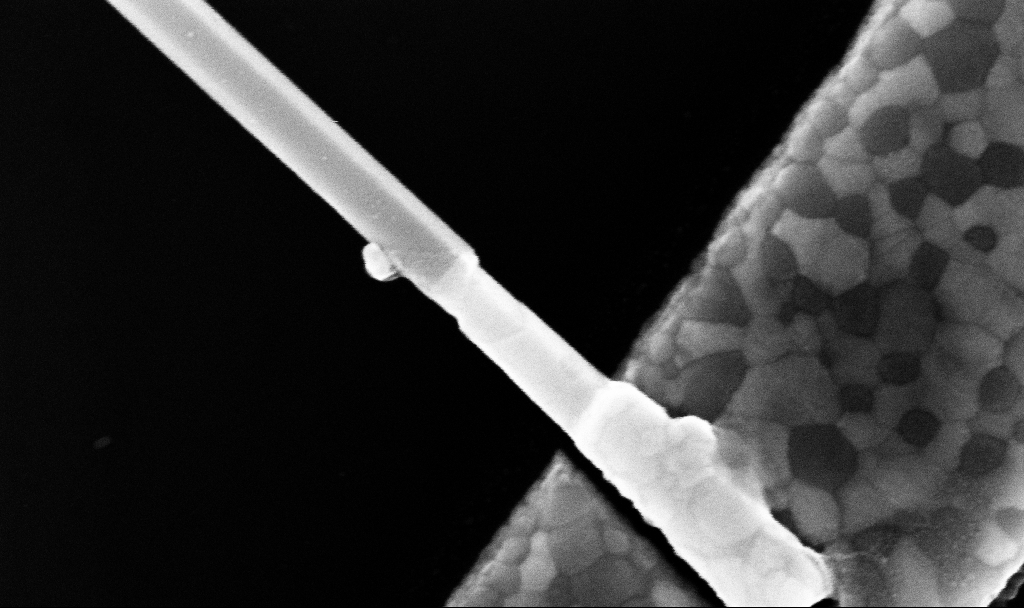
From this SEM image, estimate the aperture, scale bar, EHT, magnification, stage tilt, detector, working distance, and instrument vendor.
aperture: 30 µm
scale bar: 200 nm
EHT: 10 kV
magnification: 225.19 K X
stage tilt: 0°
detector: InLens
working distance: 7.7 mm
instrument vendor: Zeiss